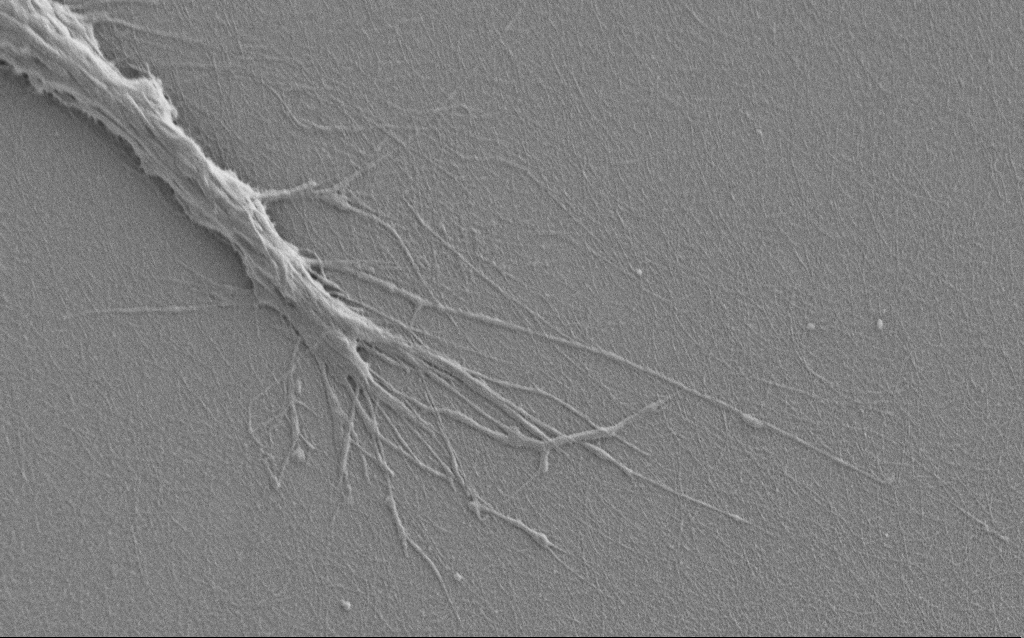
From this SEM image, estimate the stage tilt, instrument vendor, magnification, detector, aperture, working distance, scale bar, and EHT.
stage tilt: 0°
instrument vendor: Zeiss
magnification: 7.5 K X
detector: SE2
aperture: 30 µm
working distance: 6 mm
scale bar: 2000 nm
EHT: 1 kV